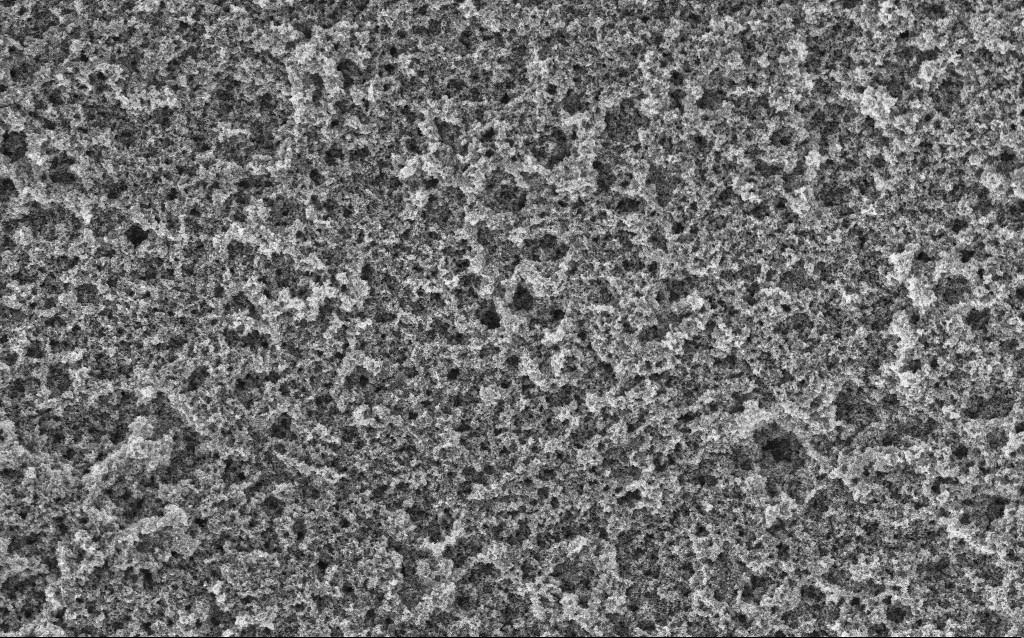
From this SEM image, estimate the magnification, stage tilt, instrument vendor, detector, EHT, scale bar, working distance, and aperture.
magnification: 15.33 K X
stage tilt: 0°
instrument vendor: Zeiss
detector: InLens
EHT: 5 kV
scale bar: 1000 nm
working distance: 4.4 mm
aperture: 30 µm